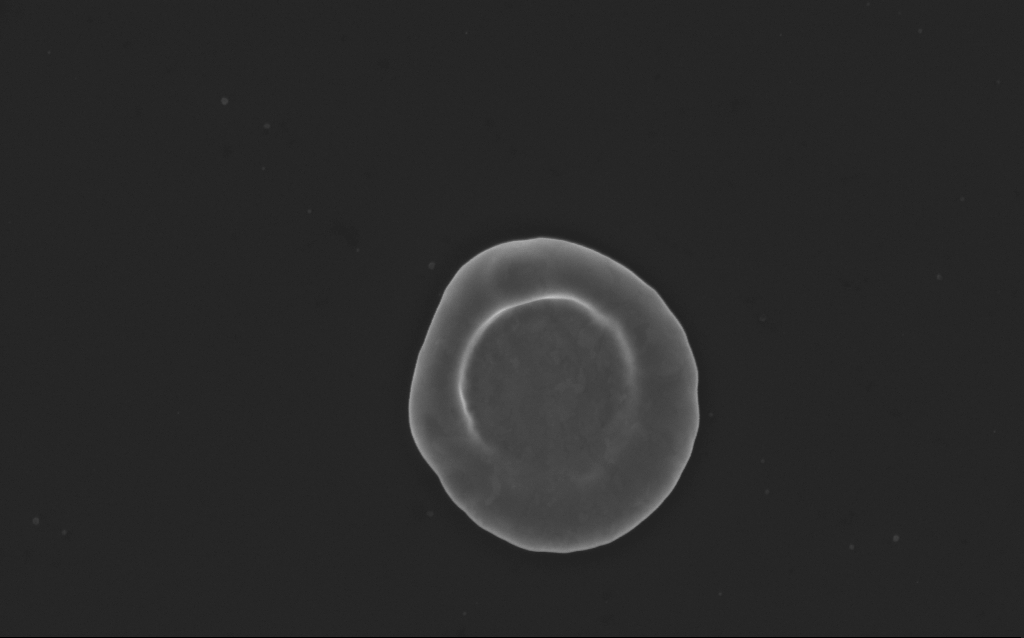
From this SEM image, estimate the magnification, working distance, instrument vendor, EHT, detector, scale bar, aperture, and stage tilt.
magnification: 49.99 K X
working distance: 4 mm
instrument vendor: Zeiss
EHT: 5 kV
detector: InLens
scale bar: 1000 nm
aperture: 30 µm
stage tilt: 0°